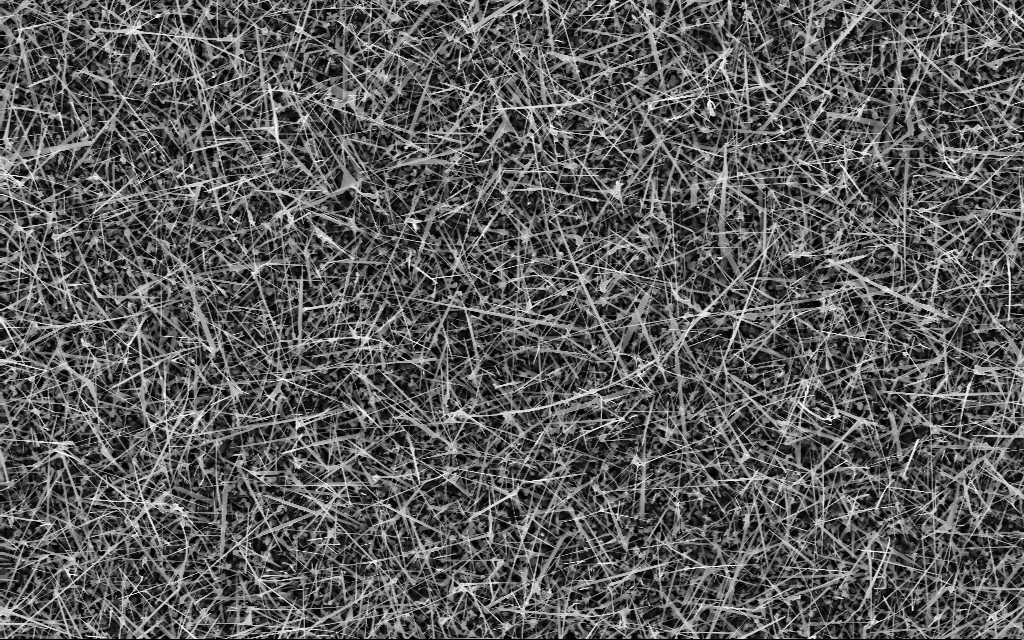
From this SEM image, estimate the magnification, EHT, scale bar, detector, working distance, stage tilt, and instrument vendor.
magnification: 10 K X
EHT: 10 kV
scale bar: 2000 nm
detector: InLens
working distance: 7 mm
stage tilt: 0°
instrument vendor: Zeiss